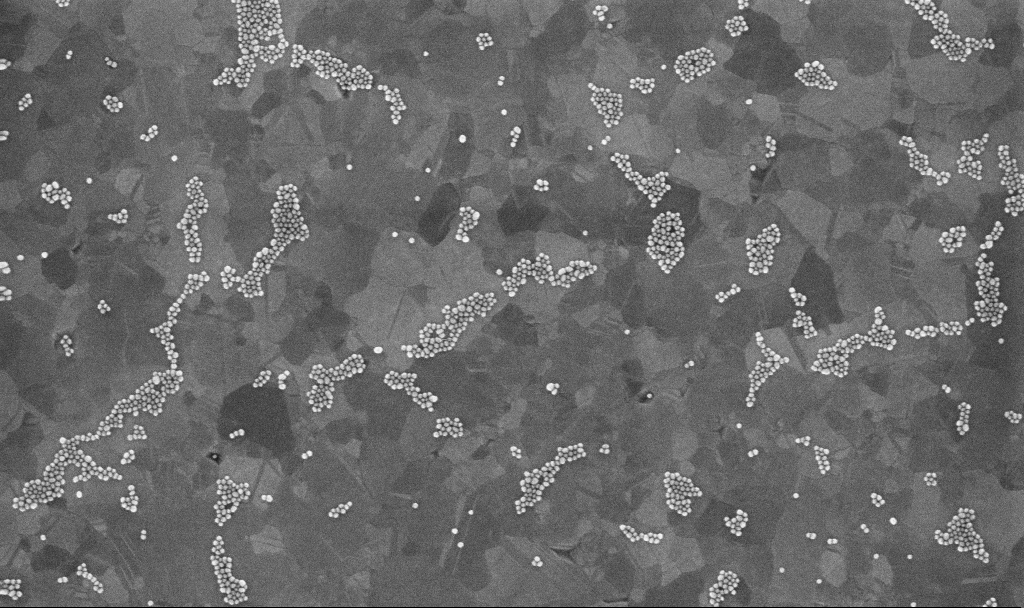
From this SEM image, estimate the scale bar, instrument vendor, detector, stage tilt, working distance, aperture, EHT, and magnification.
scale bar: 200 nm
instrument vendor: Zeiss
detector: InLens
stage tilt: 0°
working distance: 3.4 mm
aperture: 30 µm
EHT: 10 kV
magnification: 100 K X